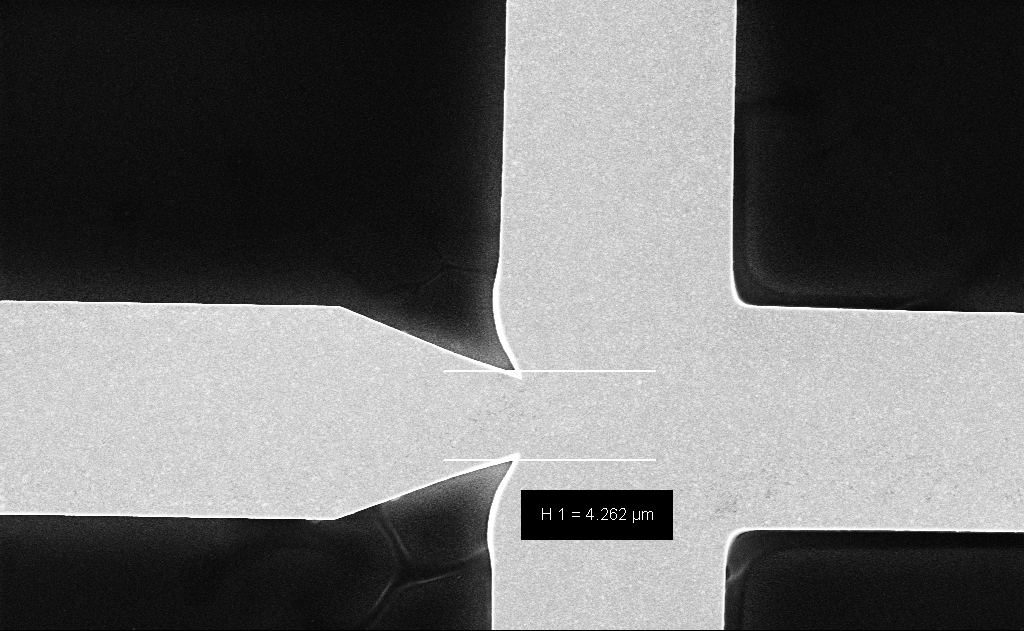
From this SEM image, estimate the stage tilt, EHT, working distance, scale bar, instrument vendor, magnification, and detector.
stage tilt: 0°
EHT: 10 kV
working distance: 12 mm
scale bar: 2000 nm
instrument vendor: Zeiss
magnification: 7.67 K X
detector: InLens